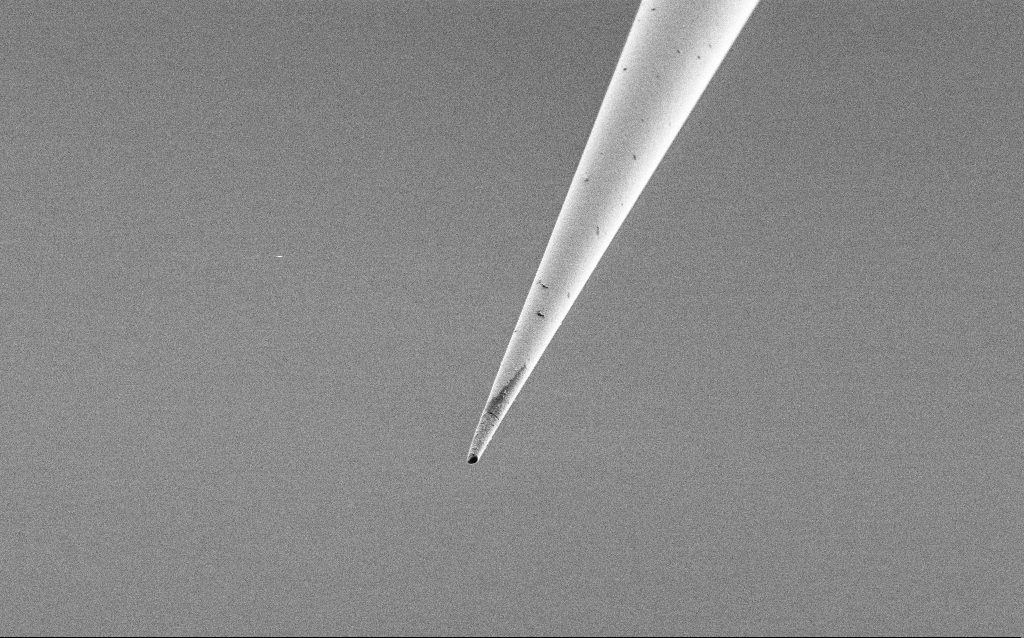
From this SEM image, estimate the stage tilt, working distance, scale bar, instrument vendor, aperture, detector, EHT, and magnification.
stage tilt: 45°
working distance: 6.7 mm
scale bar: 10000 nm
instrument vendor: Zeiss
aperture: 30 µm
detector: SE2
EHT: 1 kV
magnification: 5 K X